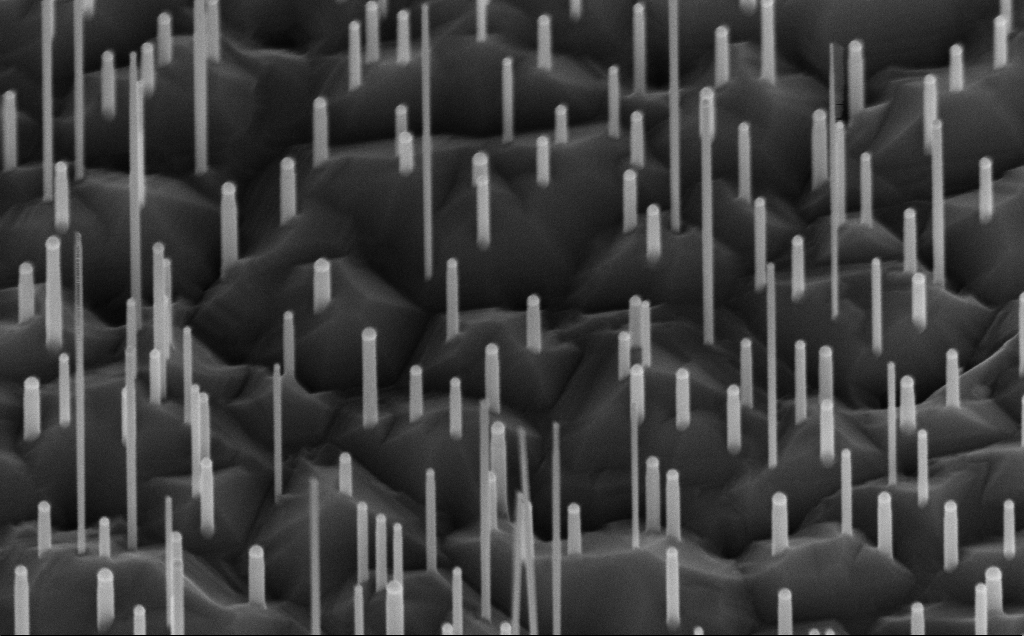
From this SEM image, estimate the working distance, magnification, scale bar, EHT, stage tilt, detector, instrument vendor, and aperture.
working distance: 7 mm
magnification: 80 K X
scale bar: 200 nm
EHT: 10 kV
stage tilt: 45°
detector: InLens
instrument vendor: Zeiss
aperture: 30 µm